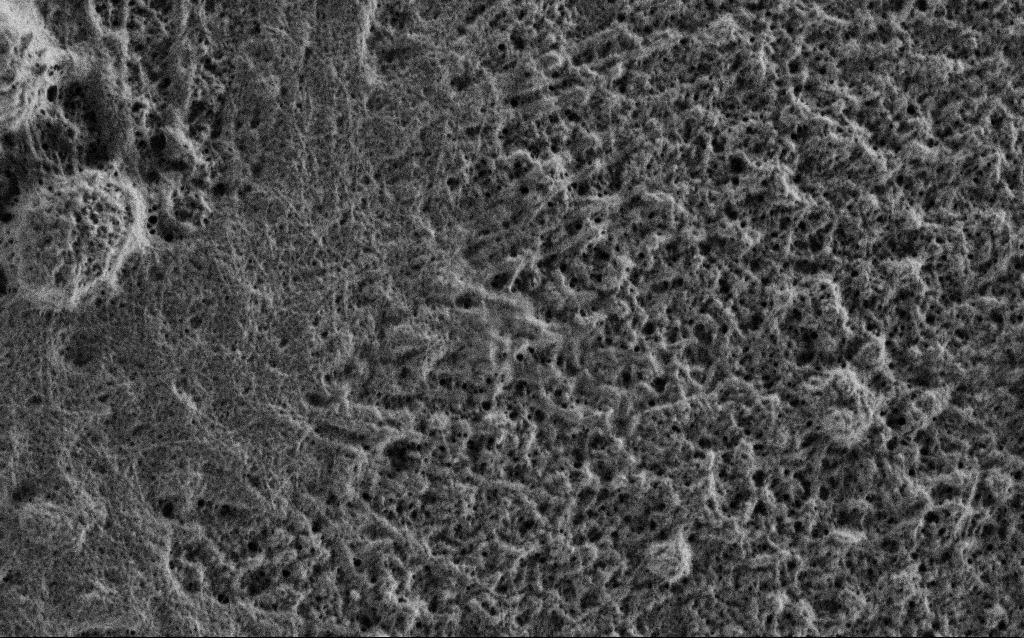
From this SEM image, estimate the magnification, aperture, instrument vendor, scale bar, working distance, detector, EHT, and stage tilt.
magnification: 25 K X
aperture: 30 µm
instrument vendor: Zeiss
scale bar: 1000 nm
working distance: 3.4 mm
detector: SE2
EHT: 0.9 kV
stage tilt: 0°